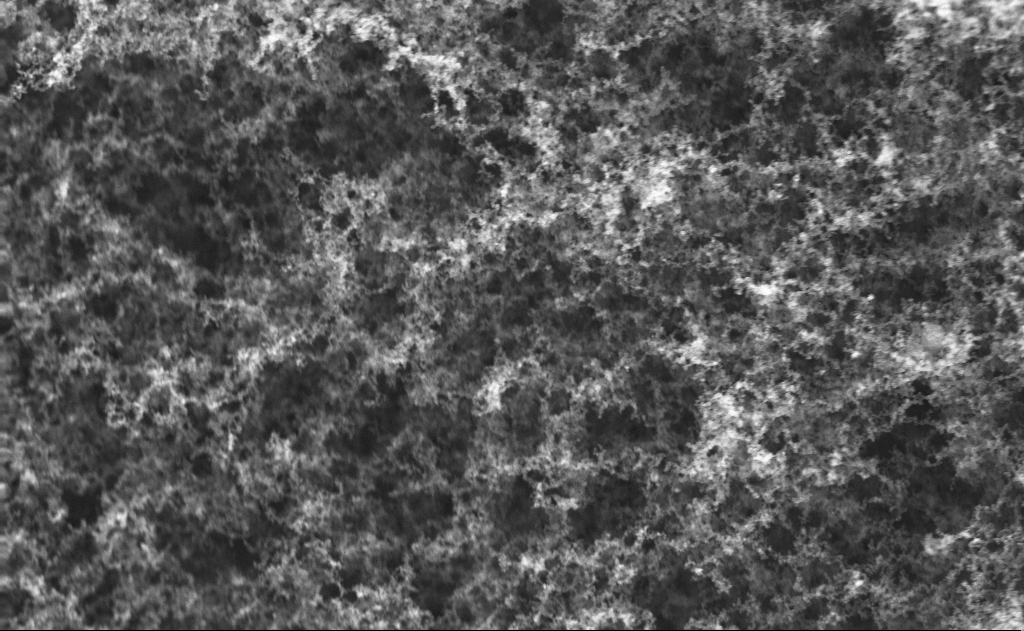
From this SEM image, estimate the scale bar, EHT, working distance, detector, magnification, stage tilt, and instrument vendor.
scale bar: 1000 nm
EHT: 10 kV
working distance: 2 mm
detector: InLens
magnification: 62.86 K X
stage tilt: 0.6°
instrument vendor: Zeiss